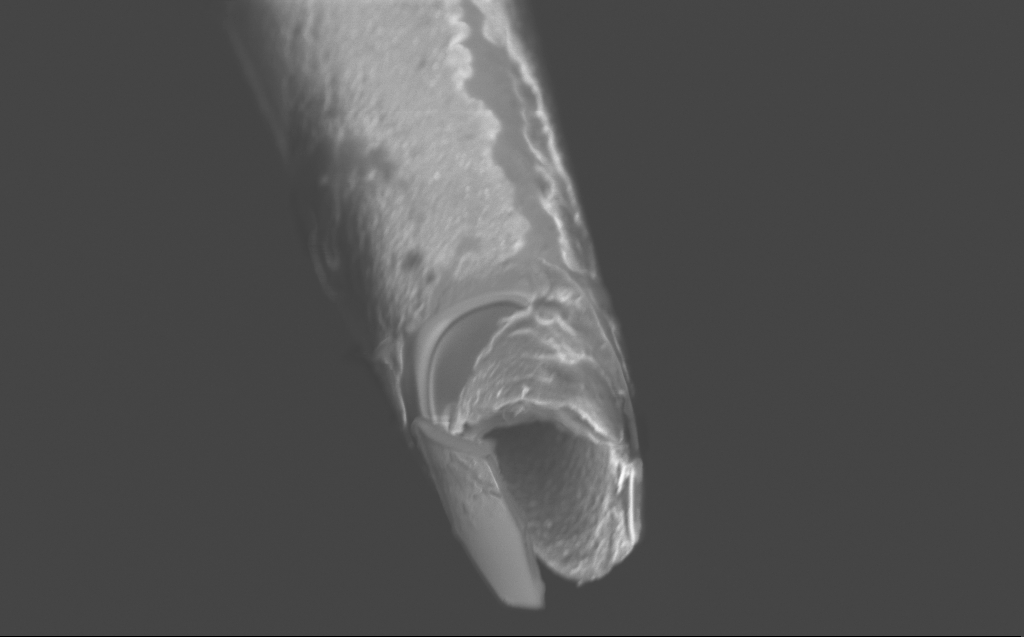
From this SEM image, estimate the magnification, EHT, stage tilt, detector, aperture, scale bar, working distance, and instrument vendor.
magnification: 21.47 K X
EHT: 3 kV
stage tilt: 45°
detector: InLens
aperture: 30 µm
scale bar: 2000 nm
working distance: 4 mm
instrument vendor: Zeiss